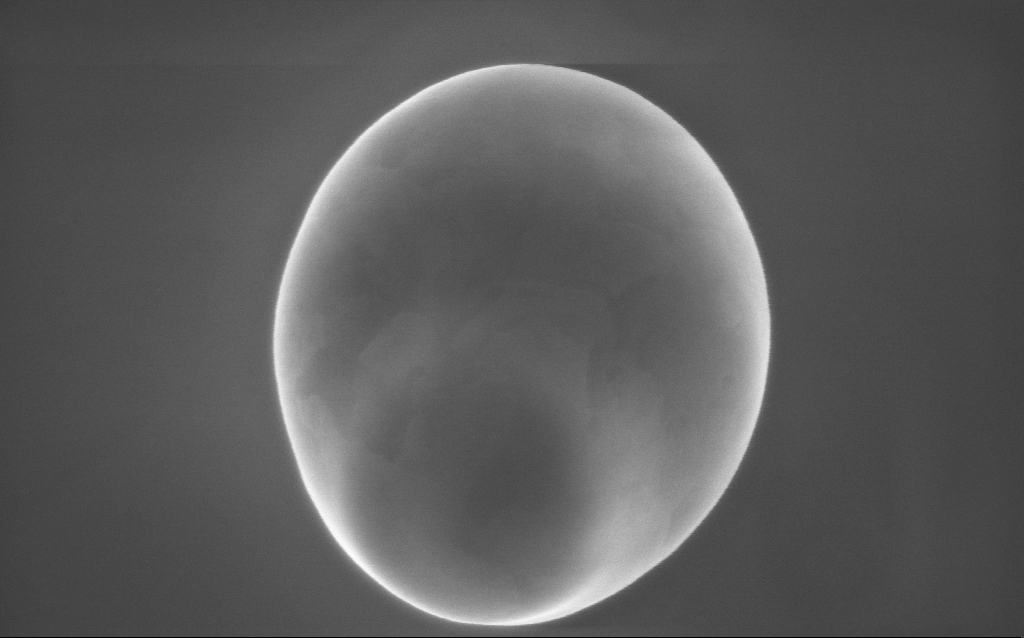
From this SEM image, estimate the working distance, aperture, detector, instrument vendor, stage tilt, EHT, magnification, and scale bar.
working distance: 2 mm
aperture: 30 µm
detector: InLens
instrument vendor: Zeiss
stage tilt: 0°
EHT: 10 kV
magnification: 71 K X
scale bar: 1000 nm